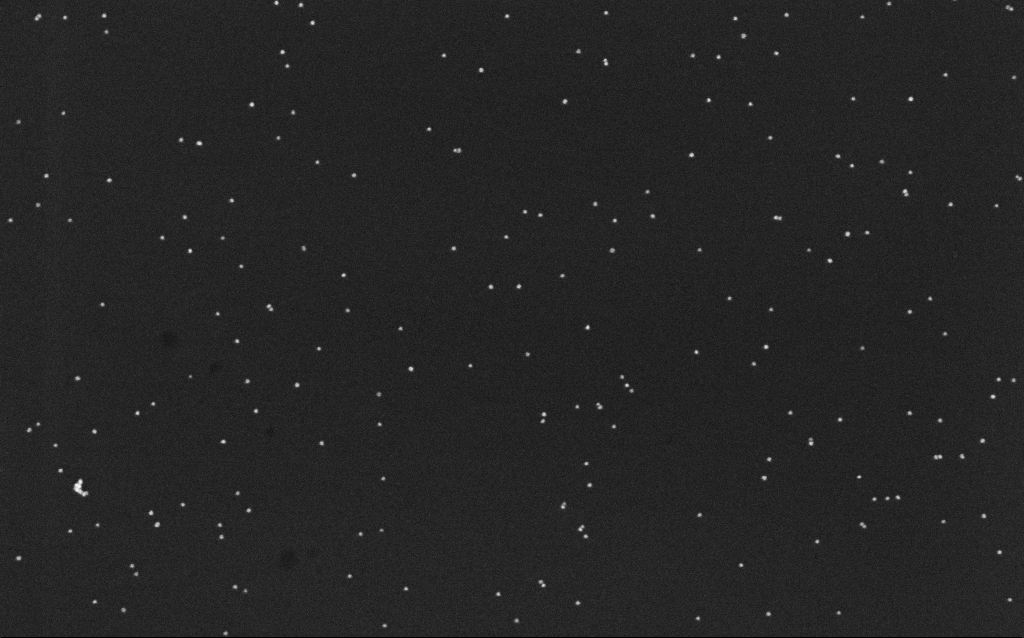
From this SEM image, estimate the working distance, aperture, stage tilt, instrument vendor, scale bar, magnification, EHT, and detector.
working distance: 6.5 mm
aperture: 30 µm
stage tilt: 0°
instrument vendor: Zeiss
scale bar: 200 nm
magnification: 100 K X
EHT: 10 kV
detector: InLens